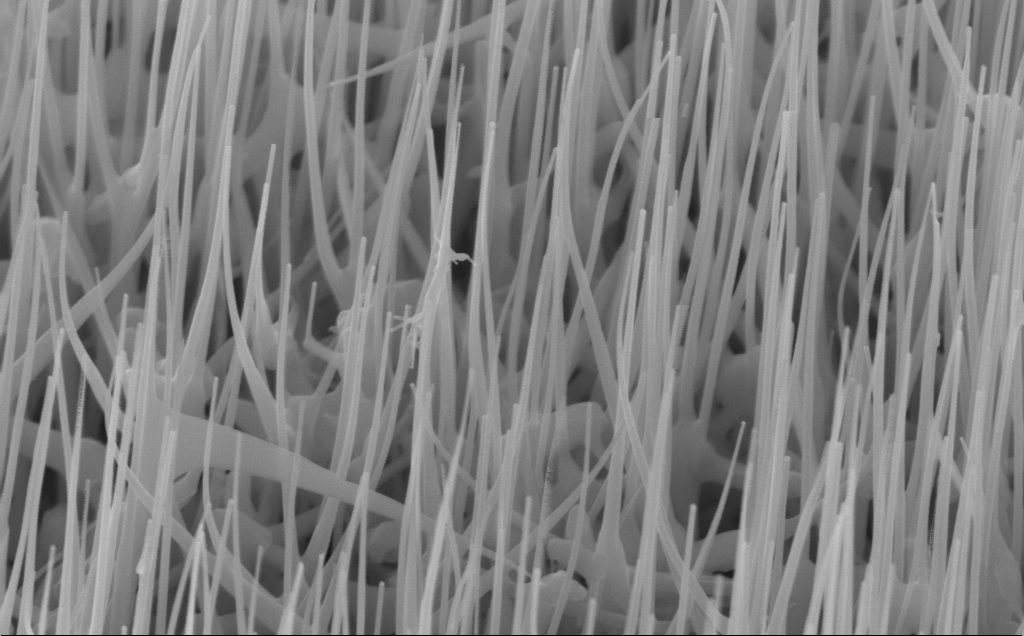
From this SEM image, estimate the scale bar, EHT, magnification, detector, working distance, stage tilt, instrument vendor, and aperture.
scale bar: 200 nm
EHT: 10 kV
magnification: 80 K X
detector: InLens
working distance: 6 mm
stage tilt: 45°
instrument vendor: Zeiss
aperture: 30 µm